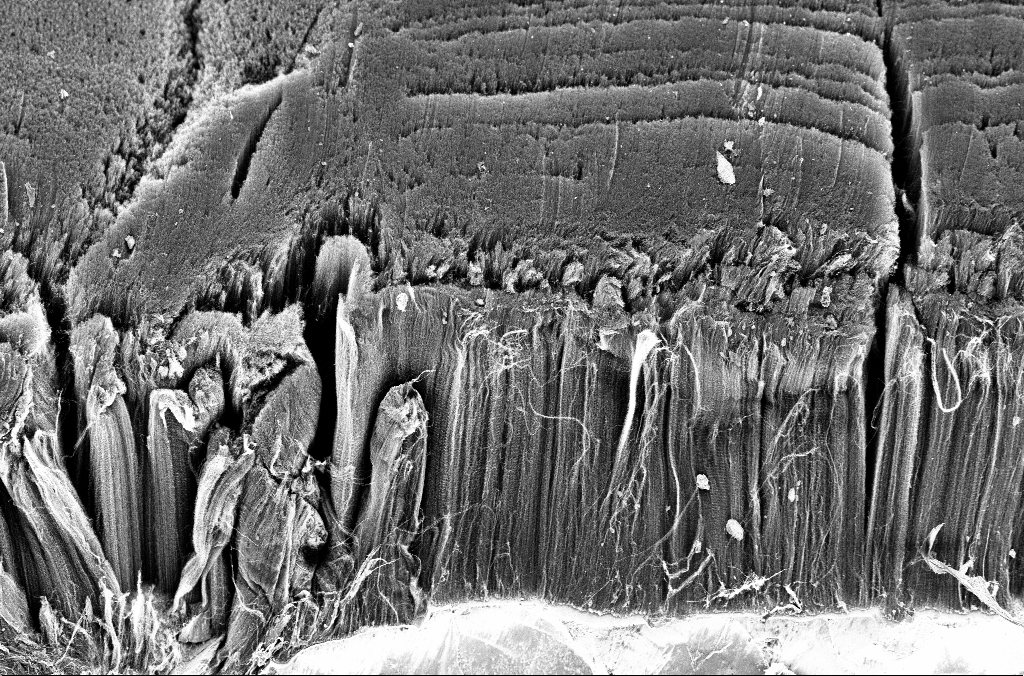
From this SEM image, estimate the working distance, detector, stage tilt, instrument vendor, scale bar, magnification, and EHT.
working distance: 3.3 mm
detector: InLens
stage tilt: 45°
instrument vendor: Zeiss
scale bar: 20000 nm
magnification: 1 K X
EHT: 3 kV